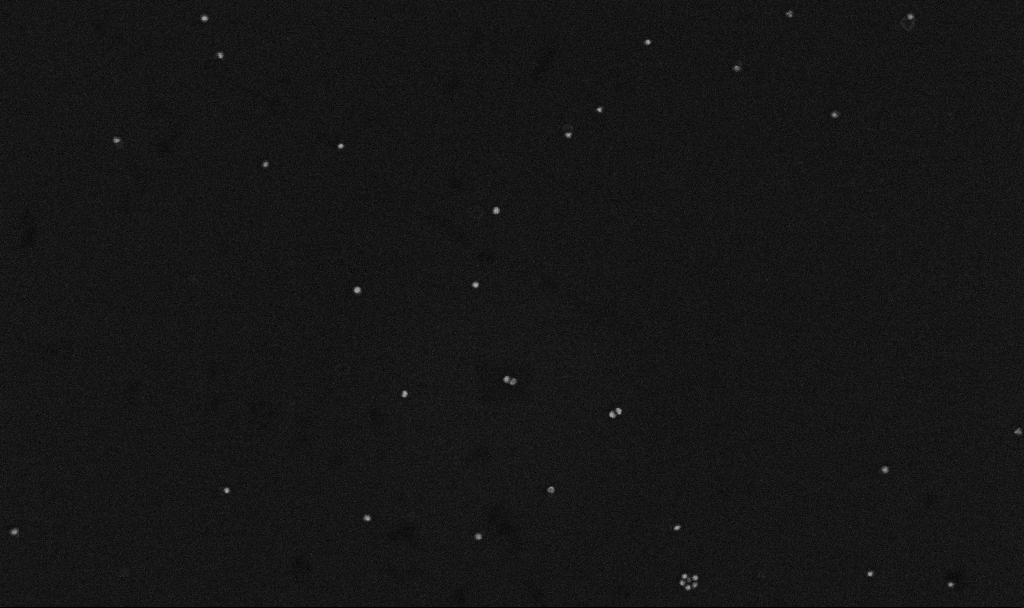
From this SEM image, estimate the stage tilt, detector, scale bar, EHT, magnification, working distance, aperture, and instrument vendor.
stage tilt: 0°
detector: InLens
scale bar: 200 nm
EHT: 10 kV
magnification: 100 K X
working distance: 3.3 mm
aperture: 30 µm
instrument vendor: Zeiss